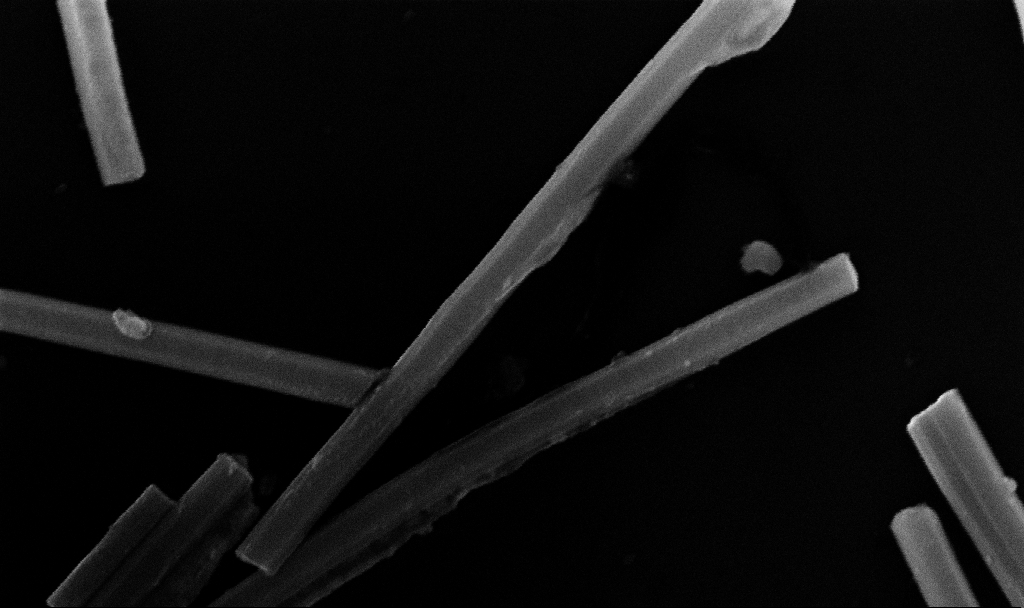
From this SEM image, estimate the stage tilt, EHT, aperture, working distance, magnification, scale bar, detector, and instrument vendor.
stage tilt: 0°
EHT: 10 kV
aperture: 30 µm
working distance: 10.7 mm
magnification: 145 K X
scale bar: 200 nm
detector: InLens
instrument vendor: Zeiss